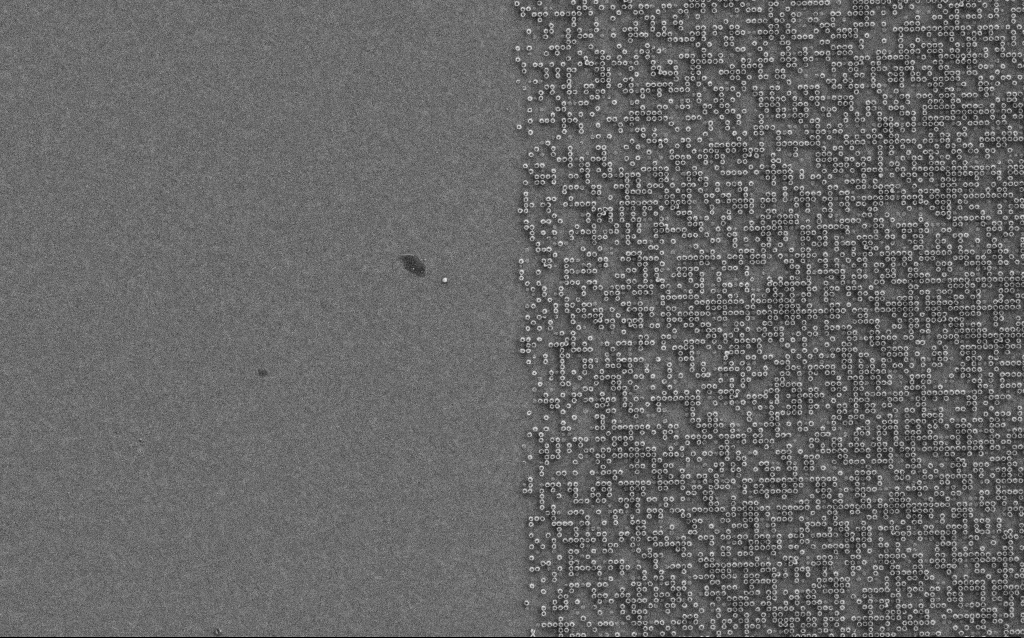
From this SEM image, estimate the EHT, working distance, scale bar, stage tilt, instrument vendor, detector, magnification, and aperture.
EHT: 1.5 kV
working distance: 5.8 mm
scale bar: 2000 nm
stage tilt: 0°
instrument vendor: Zeiss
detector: SE2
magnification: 20.59 K X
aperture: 30 µm